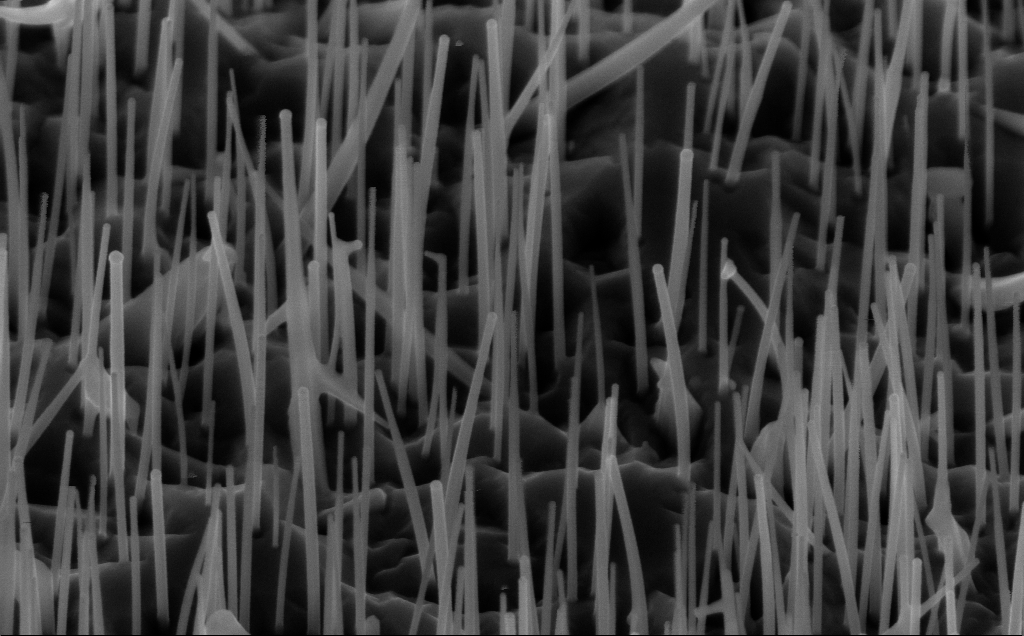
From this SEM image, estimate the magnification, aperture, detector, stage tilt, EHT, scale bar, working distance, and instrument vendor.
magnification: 80 K X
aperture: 30 µm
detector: InLens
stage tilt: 45°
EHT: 10 kV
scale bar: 200 nm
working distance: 5 mm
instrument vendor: Zeiss